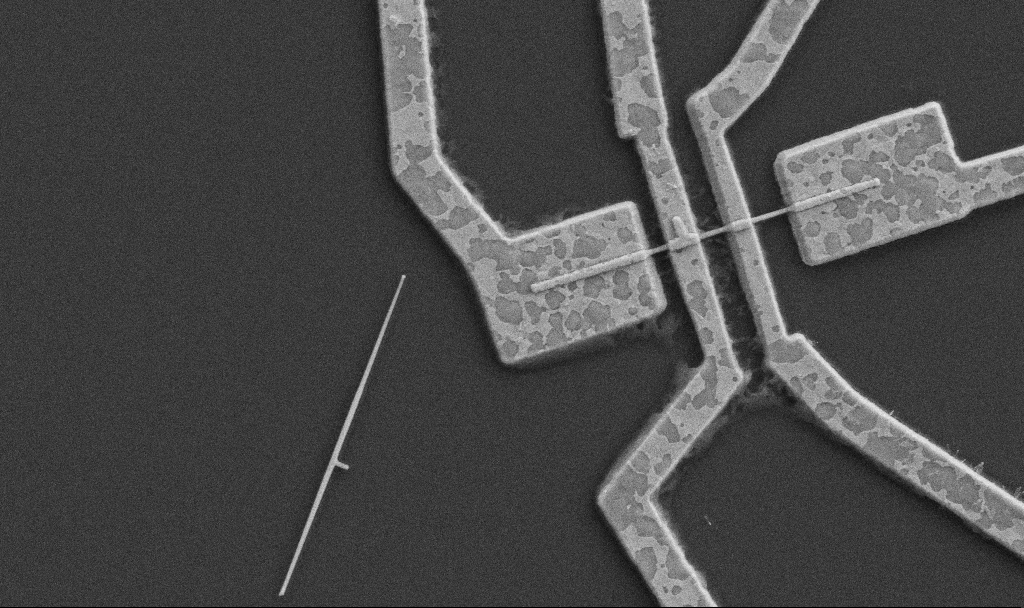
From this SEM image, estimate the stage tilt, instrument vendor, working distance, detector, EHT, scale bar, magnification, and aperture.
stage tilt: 0°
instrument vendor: Zeiss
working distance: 8.7 mm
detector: SE2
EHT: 5 kV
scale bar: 1000 nm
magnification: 20 K X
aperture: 30 µm